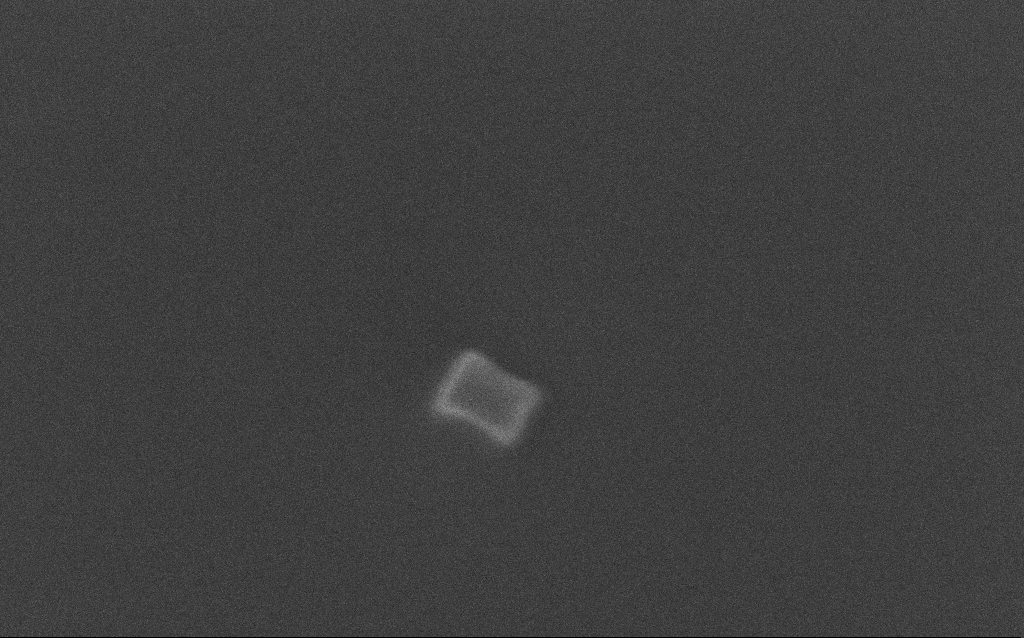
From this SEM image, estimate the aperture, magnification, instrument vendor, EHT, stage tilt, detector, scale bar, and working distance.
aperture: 30 µm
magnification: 244.72 K X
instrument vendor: Zeiss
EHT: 10 kV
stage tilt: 0°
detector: InLens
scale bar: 200 nm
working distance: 3 mm